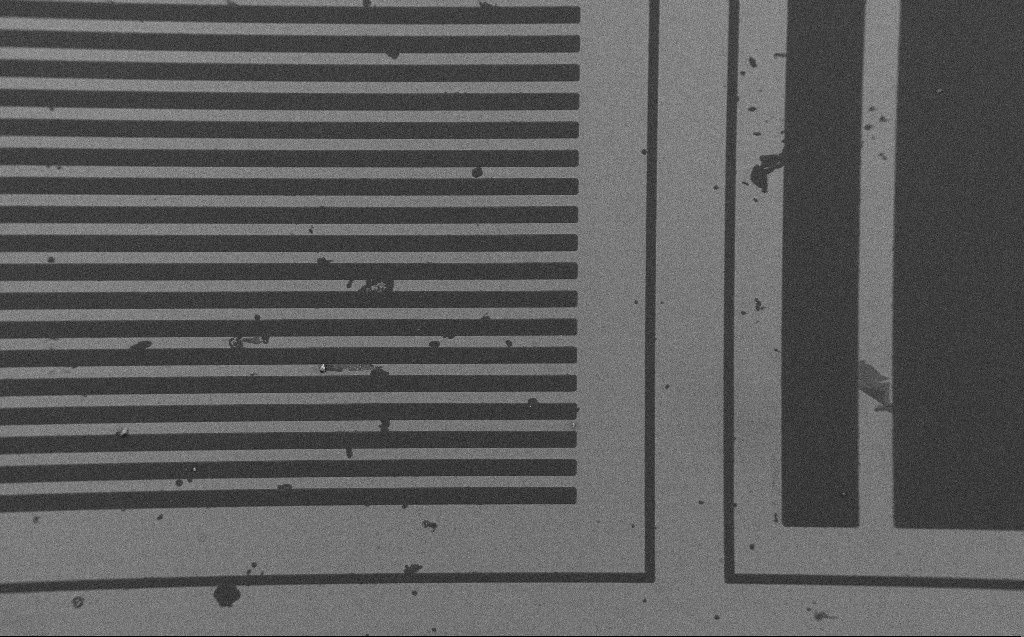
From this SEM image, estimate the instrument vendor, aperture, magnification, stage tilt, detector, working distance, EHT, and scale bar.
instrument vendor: Zeiss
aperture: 30 µm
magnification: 0.261 K X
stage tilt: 0°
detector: SE2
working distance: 4 mm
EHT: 1.2 kV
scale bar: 100000 nm